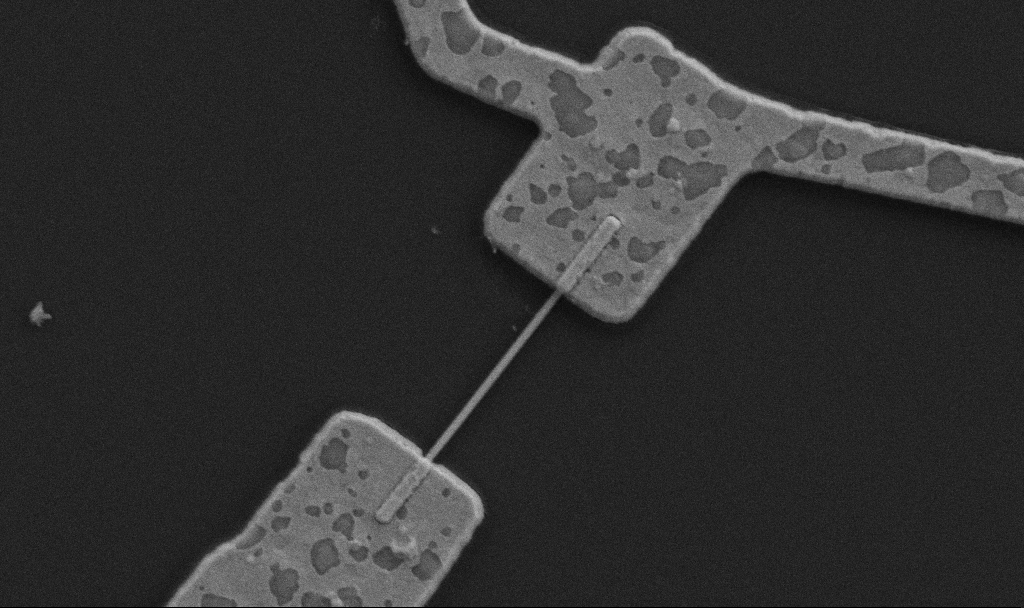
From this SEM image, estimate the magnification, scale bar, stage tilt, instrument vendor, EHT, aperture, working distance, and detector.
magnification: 30 K X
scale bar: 1000 nm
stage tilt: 0°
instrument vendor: Zeiss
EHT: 5 kV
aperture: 30 µm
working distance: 10.7 mm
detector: SE2